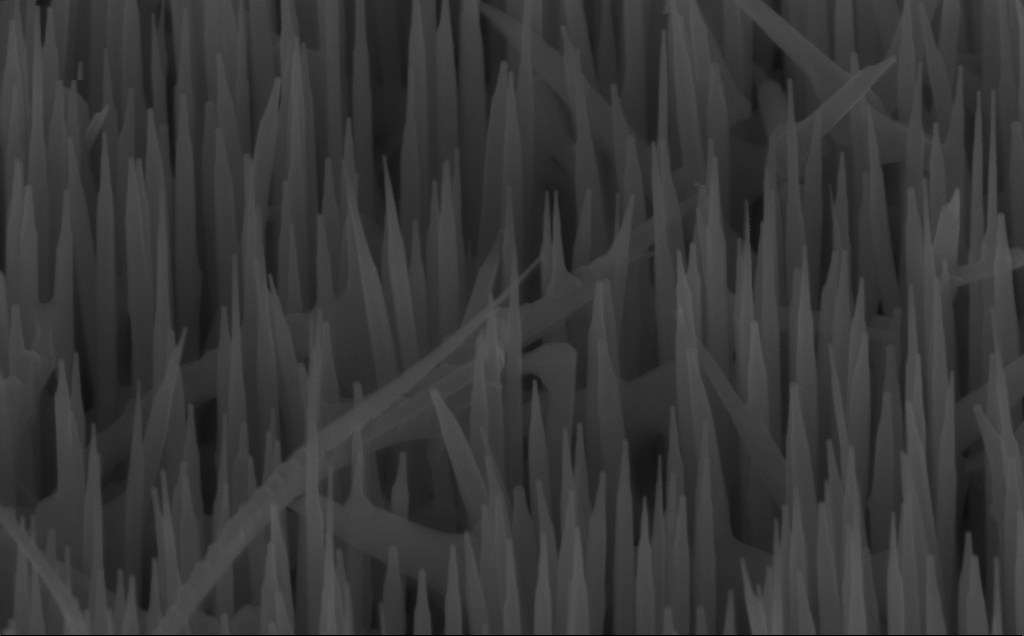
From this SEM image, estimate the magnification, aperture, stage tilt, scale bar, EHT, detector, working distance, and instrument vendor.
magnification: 80 K X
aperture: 30 µm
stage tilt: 45°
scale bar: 200 nm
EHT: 10 kV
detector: InLens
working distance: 7 mm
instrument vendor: Zeiss